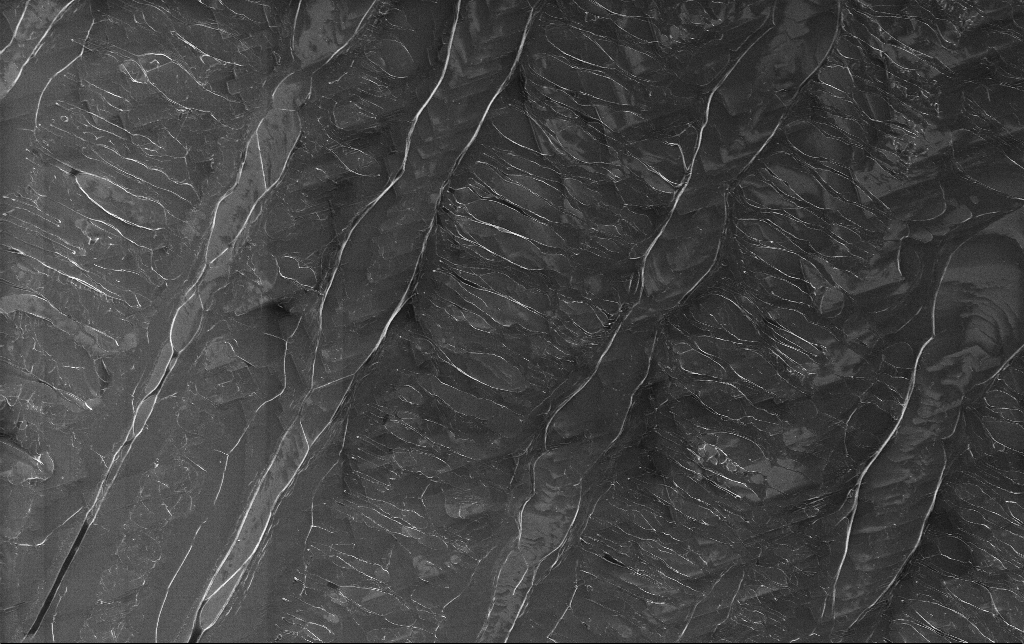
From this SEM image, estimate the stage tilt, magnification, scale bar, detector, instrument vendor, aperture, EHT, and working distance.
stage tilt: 0°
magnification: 1.28 K X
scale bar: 10000 nm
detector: InLens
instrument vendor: Zeiss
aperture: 30 µm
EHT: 5 kV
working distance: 3.1 mm